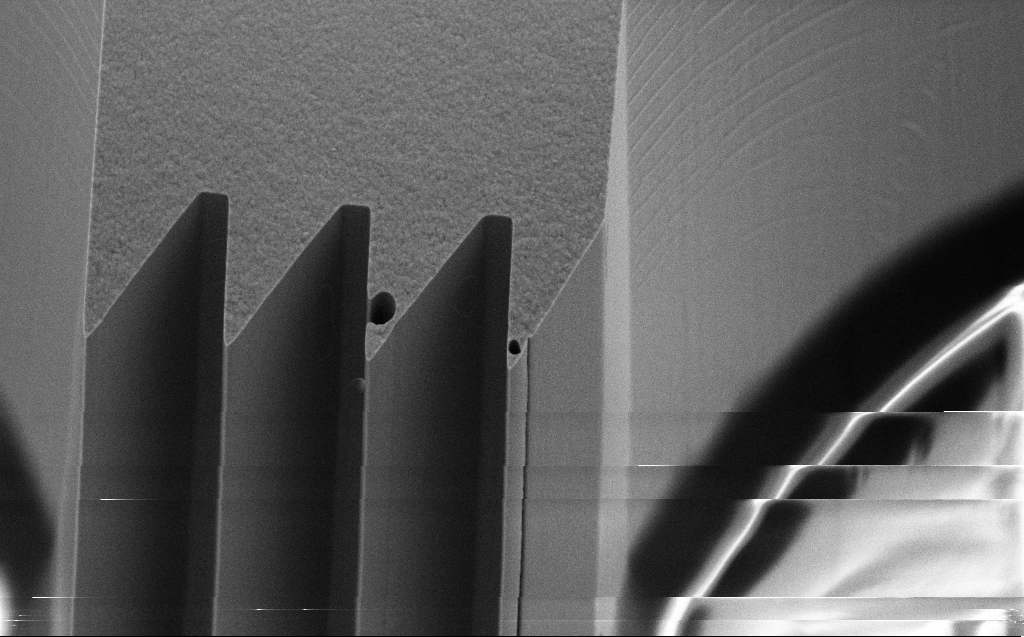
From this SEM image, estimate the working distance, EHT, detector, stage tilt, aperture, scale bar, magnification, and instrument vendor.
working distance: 7 mm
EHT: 10 kV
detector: SE2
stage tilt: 45°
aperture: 30 µm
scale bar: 10000 nm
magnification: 4.59 K X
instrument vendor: Zeiss